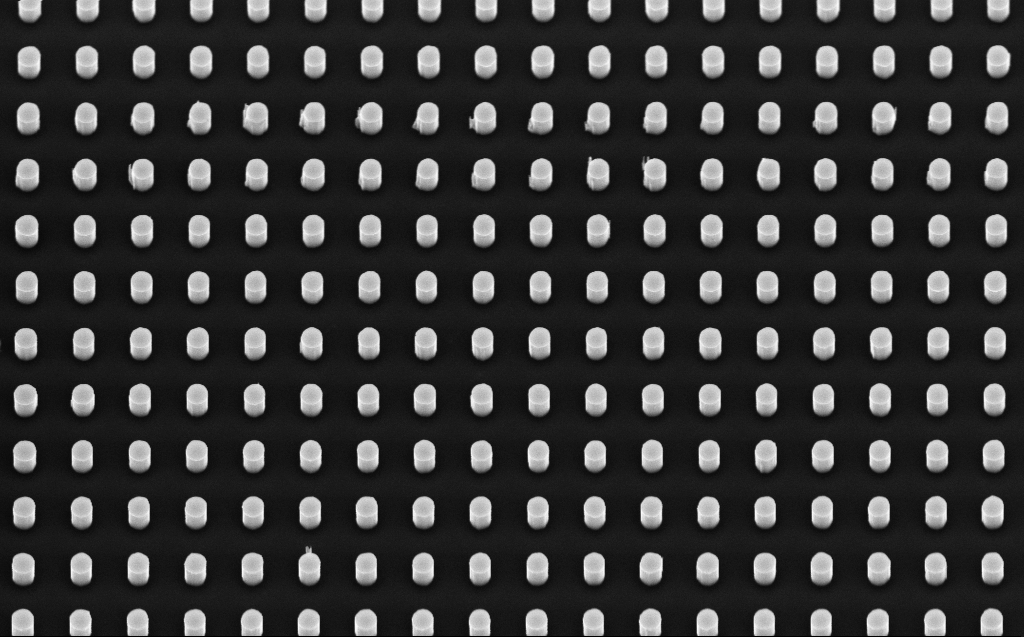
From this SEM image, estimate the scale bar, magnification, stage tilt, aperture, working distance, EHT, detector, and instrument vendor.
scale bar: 1000 nm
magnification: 20 K X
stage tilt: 30°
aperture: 30 µm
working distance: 6 mm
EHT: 5 kV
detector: InLens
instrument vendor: Zeiss